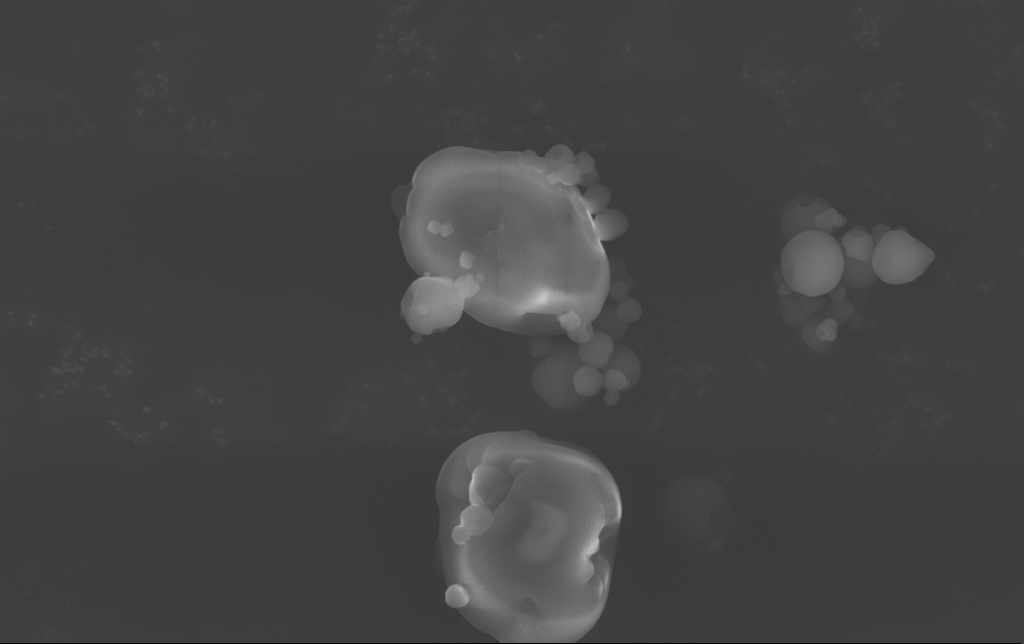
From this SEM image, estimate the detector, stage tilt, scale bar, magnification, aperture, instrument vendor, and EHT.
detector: InLens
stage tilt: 0°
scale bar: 2000 nm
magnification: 12.33 K X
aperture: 30 µm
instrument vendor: Zeiss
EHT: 15 kV